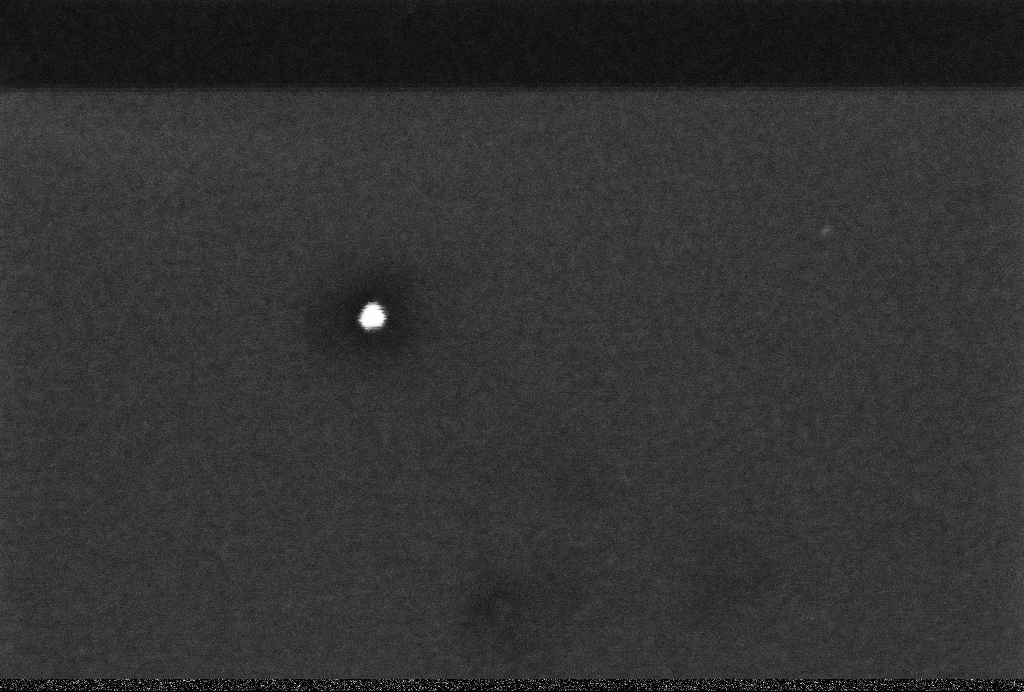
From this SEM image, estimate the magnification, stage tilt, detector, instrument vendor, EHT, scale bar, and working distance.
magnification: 368.64 K X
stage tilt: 0°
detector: InLens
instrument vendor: Zeiss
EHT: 2 kV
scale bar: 100 nm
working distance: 3.3 mm